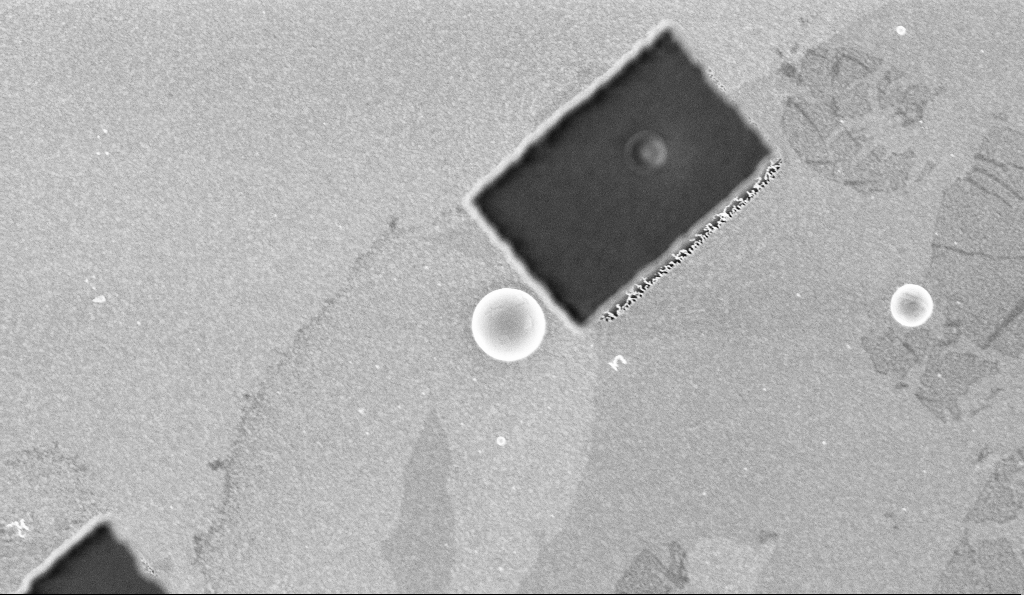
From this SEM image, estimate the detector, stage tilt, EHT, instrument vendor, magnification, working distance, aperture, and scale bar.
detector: InLens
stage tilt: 0°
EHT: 3 kV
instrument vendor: Zeiss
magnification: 22.6 K X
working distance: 5.8 mm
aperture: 30 µm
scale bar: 2000 nm